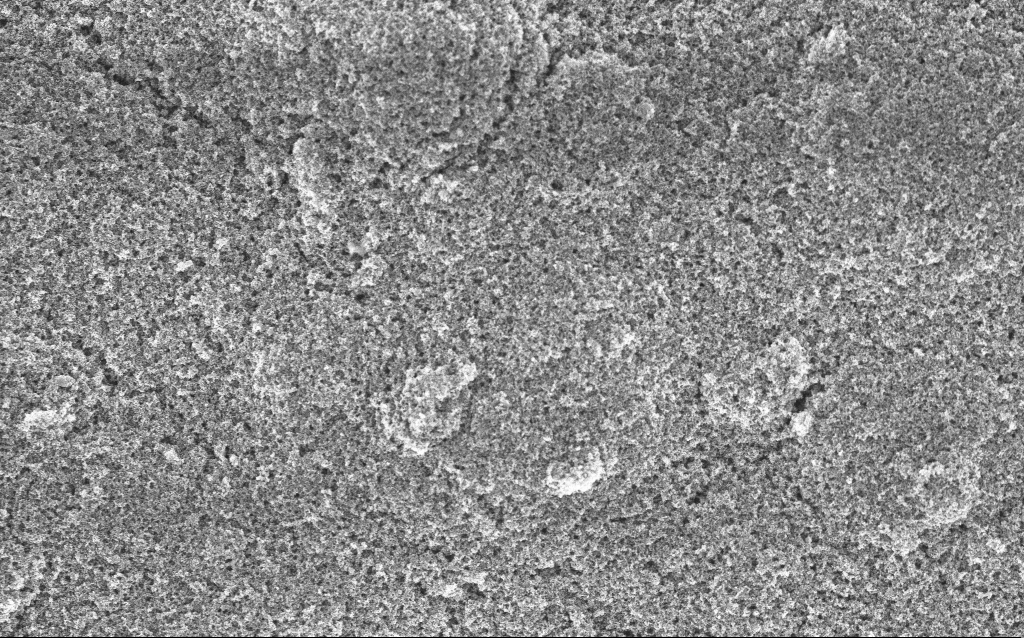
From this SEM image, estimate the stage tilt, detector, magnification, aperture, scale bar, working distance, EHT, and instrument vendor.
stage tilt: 0°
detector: InLens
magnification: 15.33 K X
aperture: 30 µm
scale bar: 1000 nm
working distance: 4.4 mm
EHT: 5 kV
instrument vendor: Zeiss